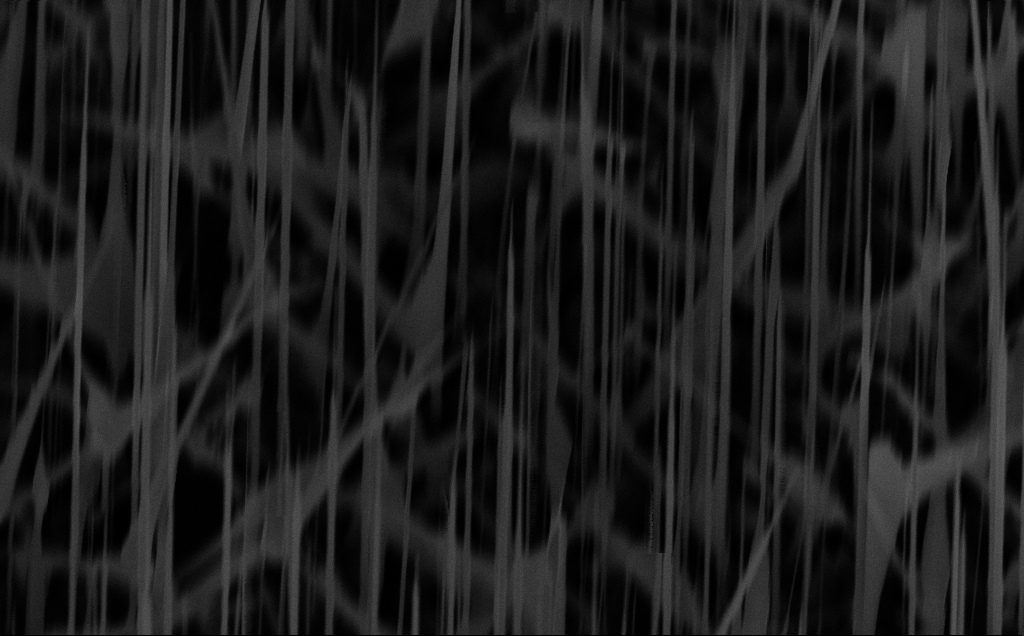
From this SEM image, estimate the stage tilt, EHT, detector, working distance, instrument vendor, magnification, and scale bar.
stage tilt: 45°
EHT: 10 kV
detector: InLens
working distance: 9 mm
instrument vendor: Zeiss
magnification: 31.26 K X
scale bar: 2000 nm